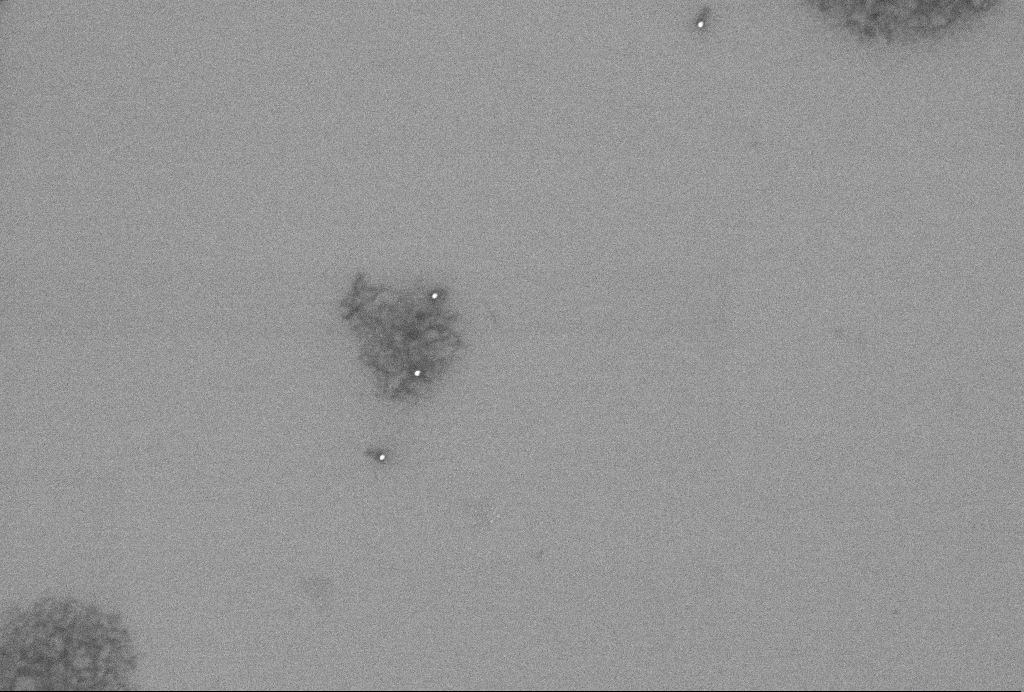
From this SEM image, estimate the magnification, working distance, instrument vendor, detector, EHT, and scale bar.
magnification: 59.14 K X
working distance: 3.3 mm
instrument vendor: Zeiss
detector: InLens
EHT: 2 kV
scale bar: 1000 nm